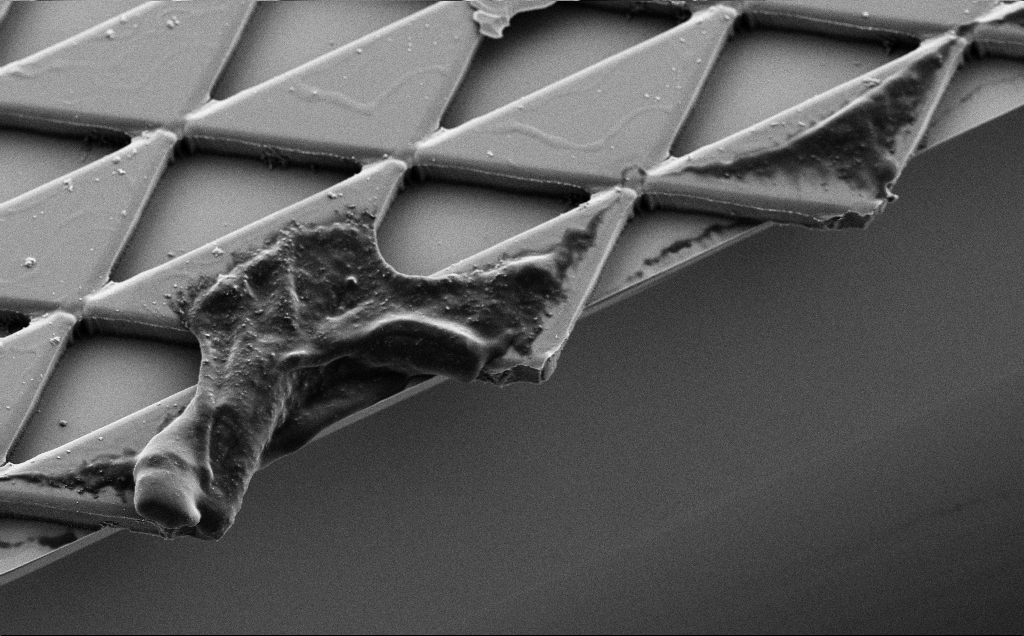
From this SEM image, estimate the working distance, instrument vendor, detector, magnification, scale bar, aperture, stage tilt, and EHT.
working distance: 8 mm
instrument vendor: Zeiss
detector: SE2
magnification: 1.46 K X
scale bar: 20000 nm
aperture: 30 µm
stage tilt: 30°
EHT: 5 kV